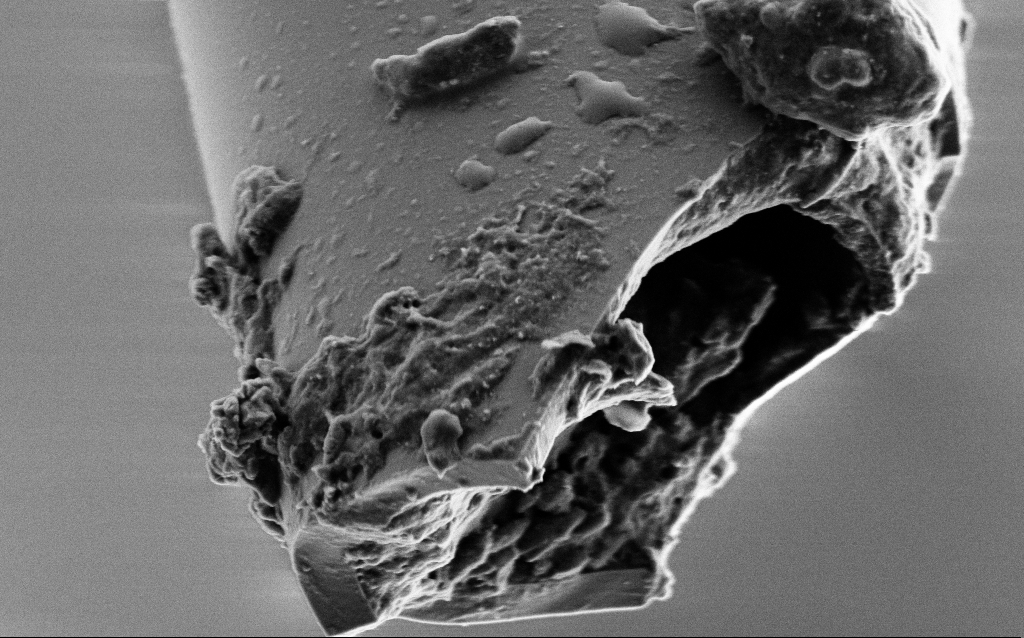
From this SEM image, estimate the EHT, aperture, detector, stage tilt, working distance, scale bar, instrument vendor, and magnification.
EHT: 2 kV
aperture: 30 µm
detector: SE2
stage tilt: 45°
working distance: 6 mm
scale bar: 2000 nm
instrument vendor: Zeiss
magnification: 25 K X